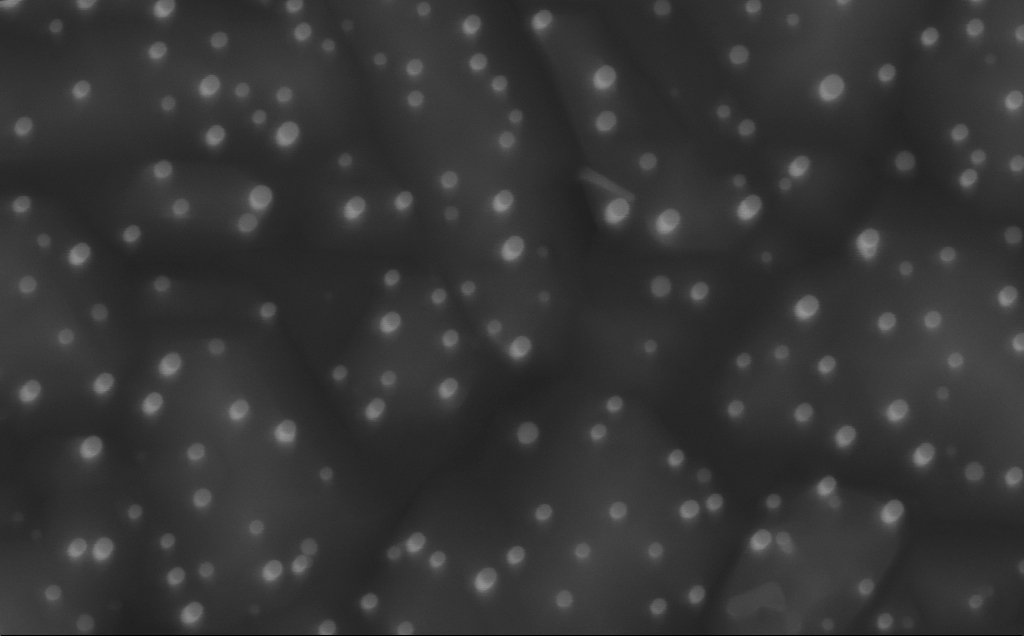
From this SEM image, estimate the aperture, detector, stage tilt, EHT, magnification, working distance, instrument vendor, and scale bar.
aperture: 30 µm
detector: InLens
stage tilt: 0°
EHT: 10 kV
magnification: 150 K X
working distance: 5 mm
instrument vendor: Zeiss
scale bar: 100 nm